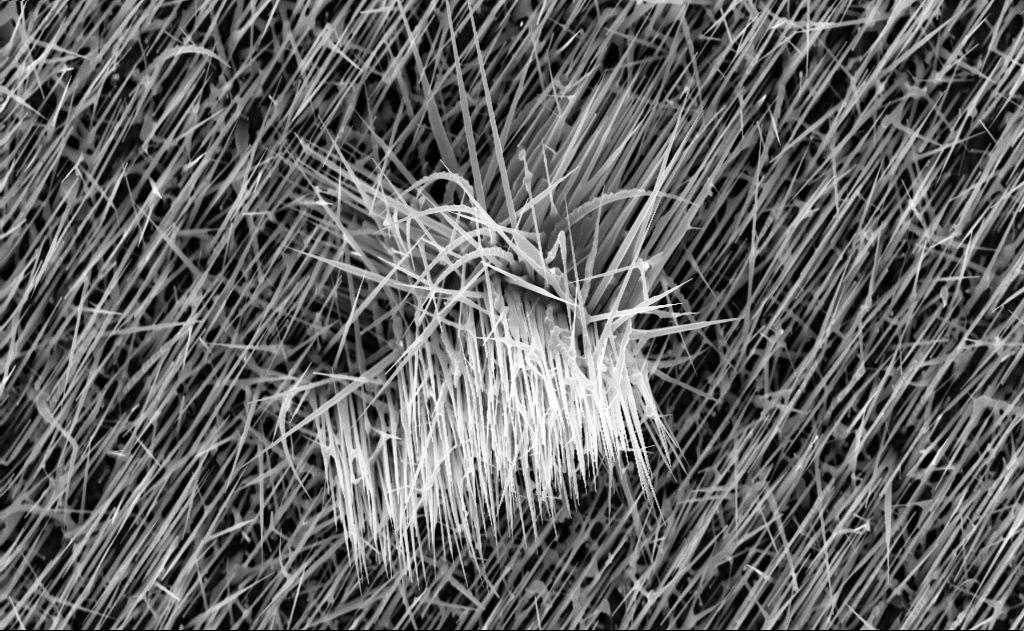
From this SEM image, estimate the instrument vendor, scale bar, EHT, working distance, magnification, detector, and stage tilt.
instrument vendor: Zeiss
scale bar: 2000 nm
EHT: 10 kV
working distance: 7 mm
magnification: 15.05 K X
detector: InLens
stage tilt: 0°